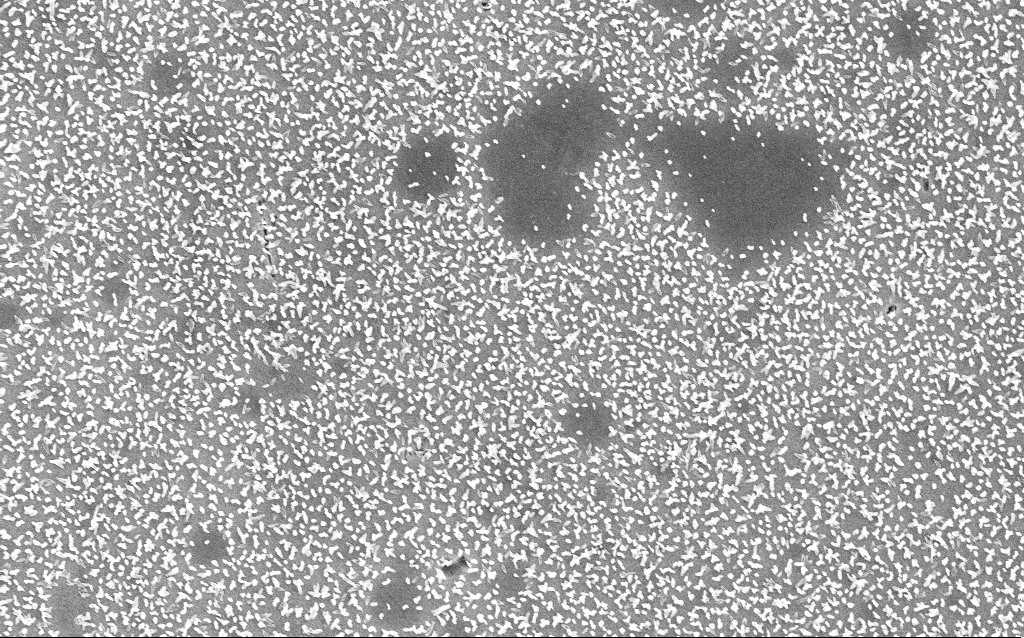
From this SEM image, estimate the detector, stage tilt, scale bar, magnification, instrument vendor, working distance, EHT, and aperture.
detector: InLens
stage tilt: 0°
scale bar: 2000 nm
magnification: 10.27 K X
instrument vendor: Zeiss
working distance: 5 mm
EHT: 6 kV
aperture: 30 µm